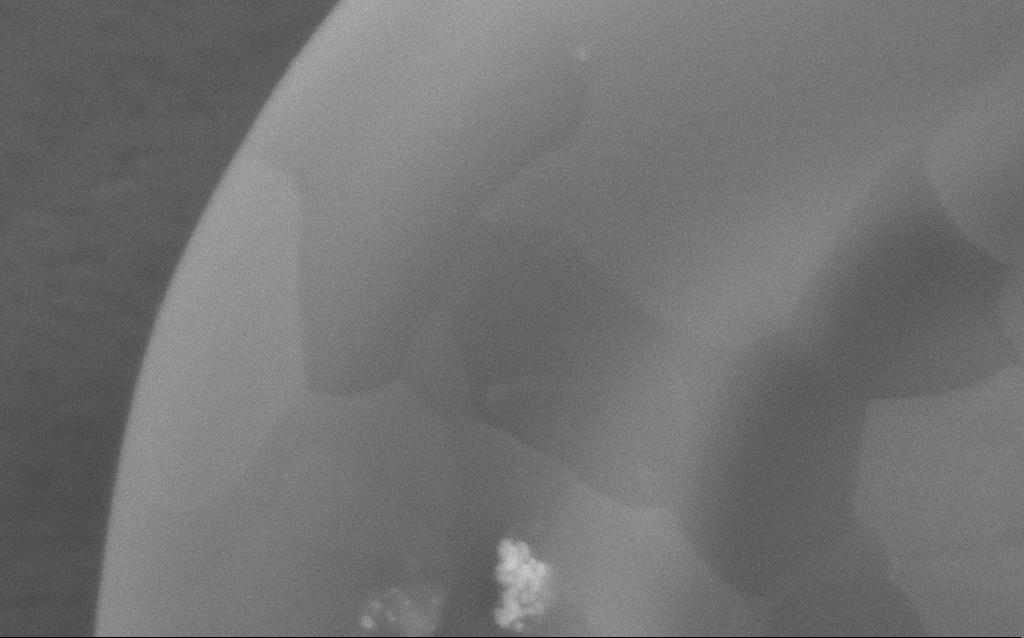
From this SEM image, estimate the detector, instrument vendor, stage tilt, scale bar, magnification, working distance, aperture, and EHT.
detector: SE2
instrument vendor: Zeiss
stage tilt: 0°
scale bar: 200 nm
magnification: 203.5 K X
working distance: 4 mm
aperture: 30 µm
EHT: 5 kV